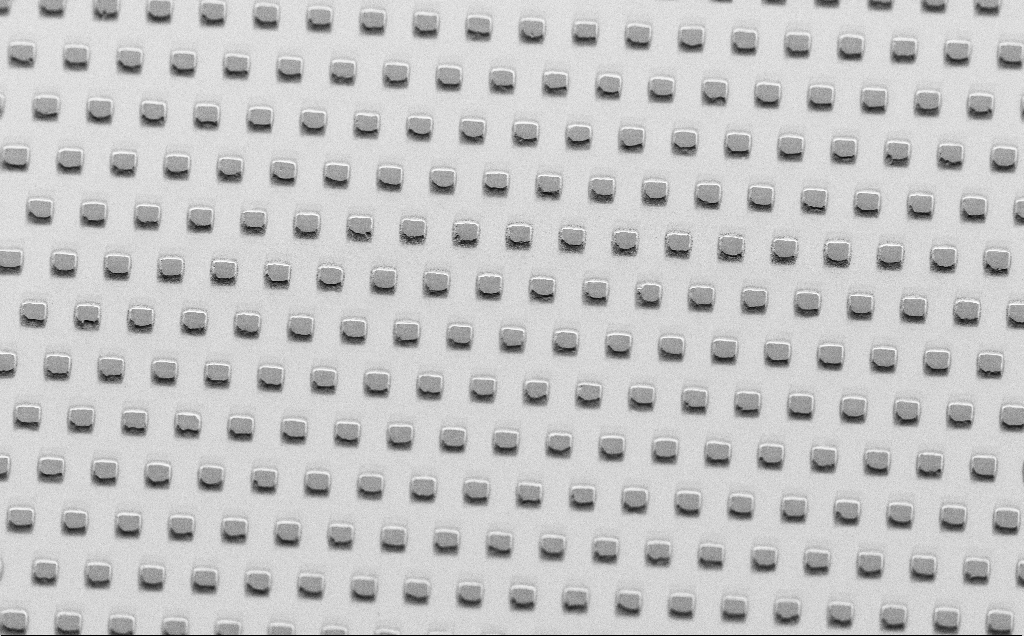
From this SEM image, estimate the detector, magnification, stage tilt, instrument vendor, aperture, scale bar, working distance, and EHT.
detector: SE2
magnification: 0.976 K X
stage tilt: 45°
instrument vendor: Zeiss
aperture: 30 µm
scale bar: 20000 nm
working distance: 7 mm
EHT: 1.5 kV